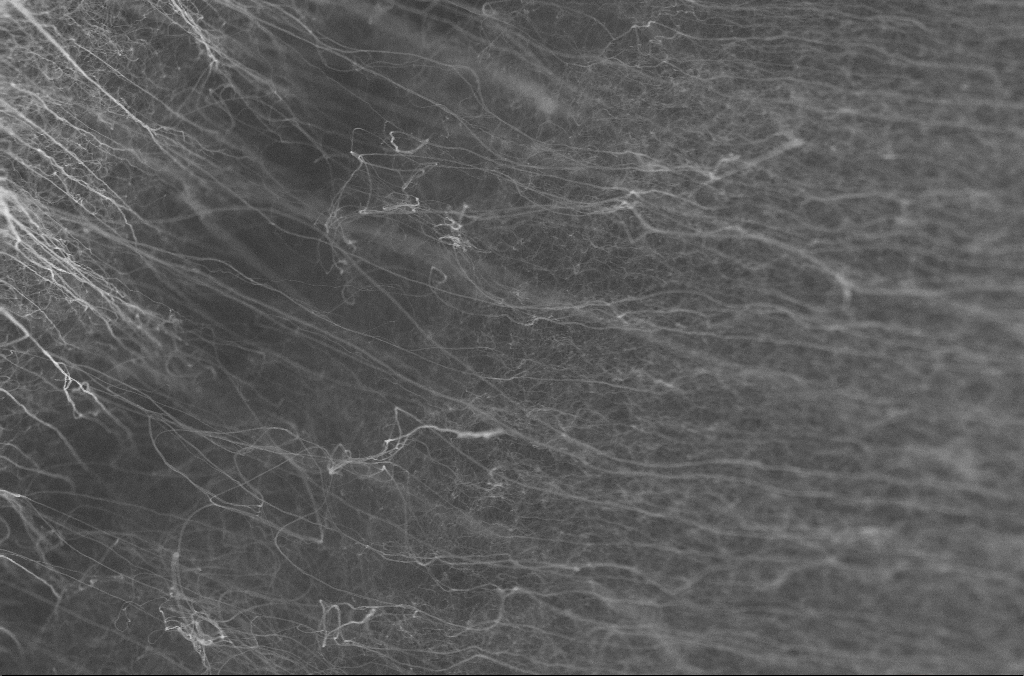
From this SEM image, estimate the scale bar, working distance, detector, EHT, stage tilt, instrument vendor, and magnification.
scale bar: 1000 nm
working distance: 4 mm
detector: InLens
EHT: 10 kV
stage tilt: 0°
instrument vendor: Zeiss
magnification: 30 K X